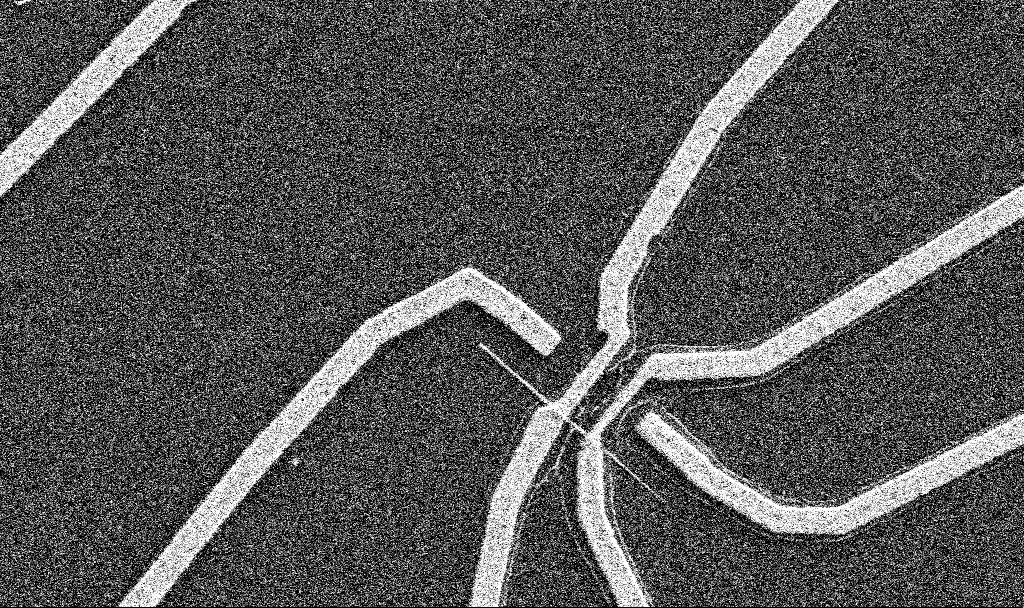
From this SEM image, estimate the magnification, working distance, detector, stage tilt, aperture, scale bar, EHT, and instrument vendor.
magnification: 14.62 K X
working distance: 10.7 mm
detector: SE2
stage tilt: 0°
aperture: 30 µm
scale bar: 2000 nm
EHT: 5 kV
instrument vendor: Zeiss